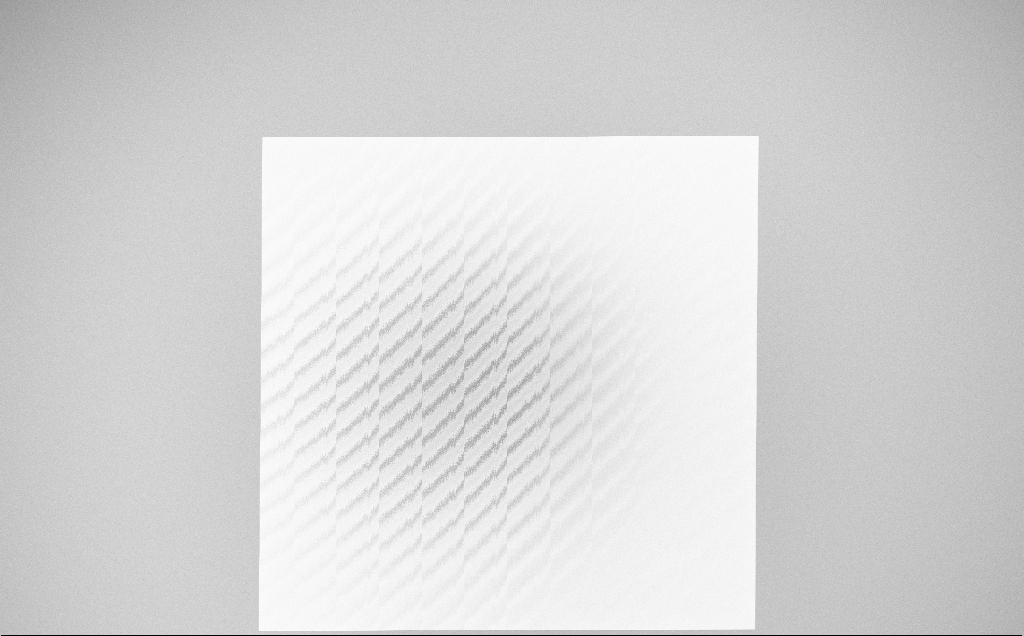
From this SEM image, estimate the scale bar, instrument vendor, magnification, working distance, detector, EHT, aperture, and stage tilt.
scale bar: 100000 nm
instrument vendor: Zeiss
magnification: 0.183 K X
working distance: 6 mm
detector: InLens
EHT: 10 kV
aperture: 30 µm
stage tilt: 0°